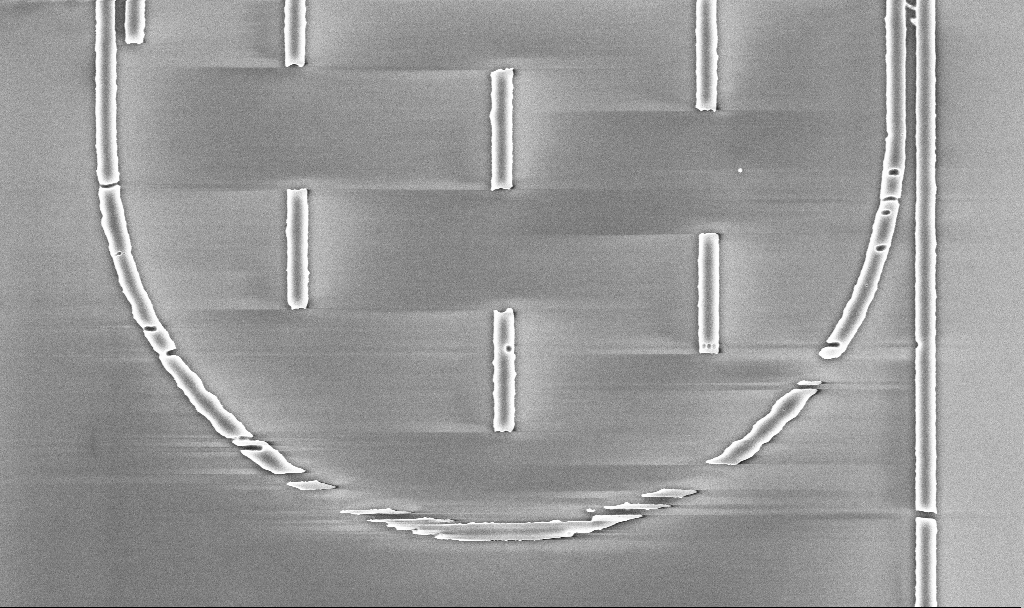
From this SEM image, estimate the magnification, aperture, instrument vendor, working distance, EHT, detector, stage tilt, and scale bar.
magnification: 15.02 K X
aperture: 30 µm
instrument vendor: Zeiss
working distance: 5.2 mm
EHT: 5 kV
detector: InLens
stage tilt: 0°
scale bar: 2000 nm